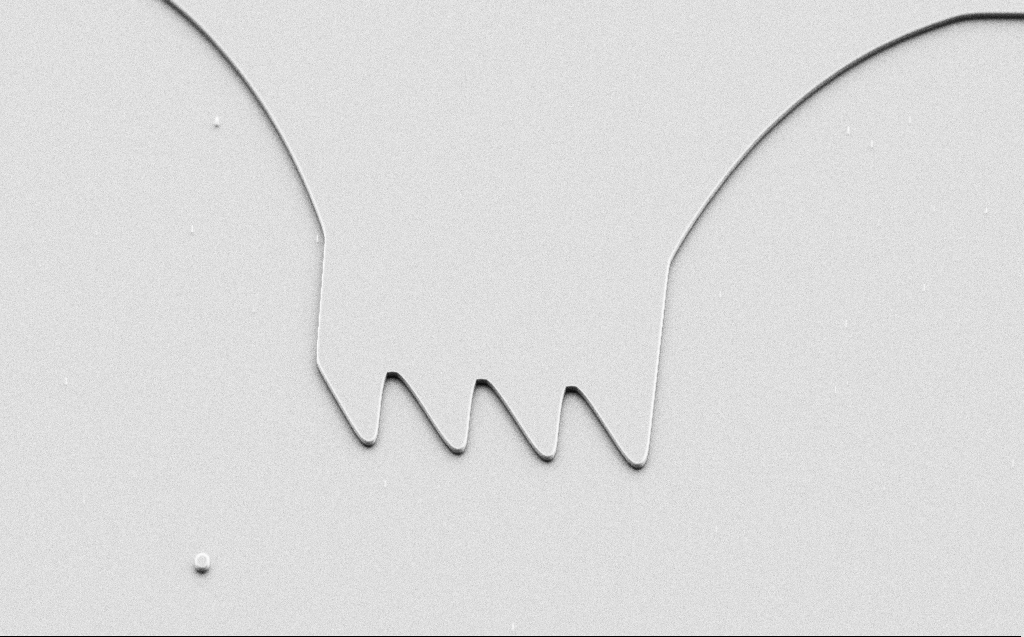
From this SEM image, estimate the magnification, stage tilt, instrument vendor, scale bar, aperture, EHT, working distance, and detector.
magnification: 2.93 K X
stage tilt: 45°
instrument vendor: Zeiss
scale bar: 10000 nm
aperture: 30 µm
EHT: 5 kV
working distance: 5 mm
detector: SE2